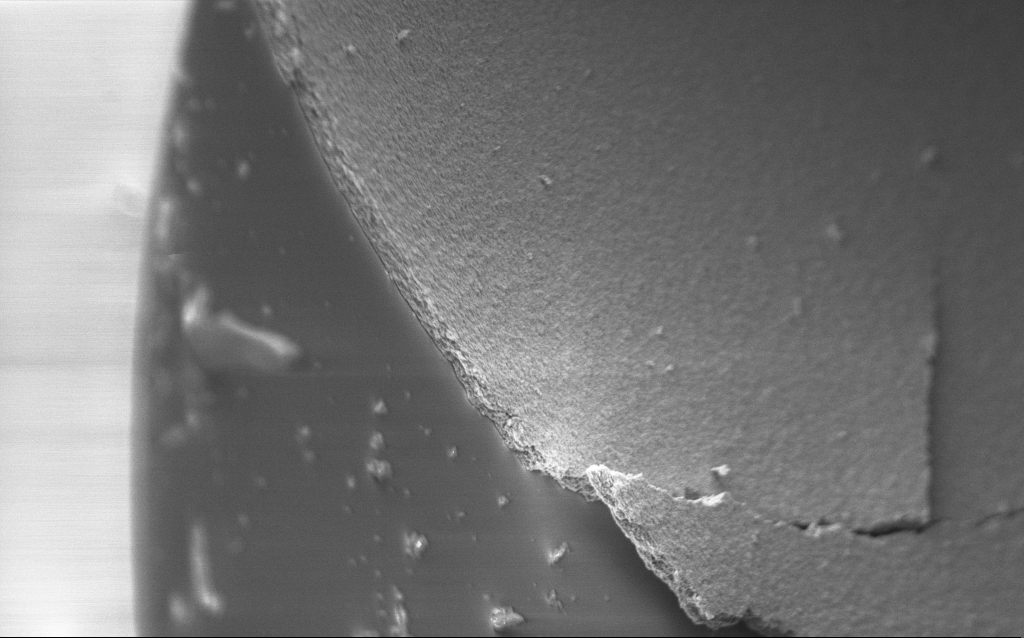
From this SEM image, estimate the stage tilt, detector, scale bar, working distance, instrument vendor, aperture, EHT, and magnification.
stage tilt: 45°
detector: InLens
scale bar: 2000 nm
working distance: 5 mm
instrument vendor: Zeiss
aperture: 30 µm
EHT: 1 kV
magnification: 22.27 K X